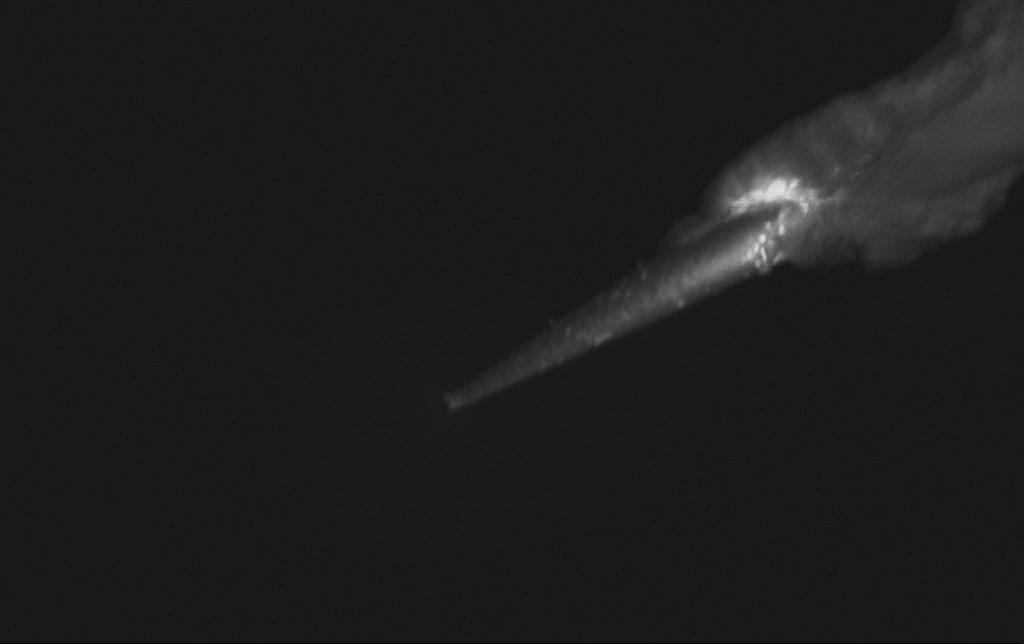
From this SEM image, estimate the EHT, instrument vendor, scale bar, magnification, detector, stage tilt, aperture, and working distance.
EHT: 3 kV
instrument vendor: Zeiss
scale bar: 2000 nm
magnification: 15 K X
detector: InLens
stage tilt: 0°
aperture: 30 µm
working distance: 6.4 mm